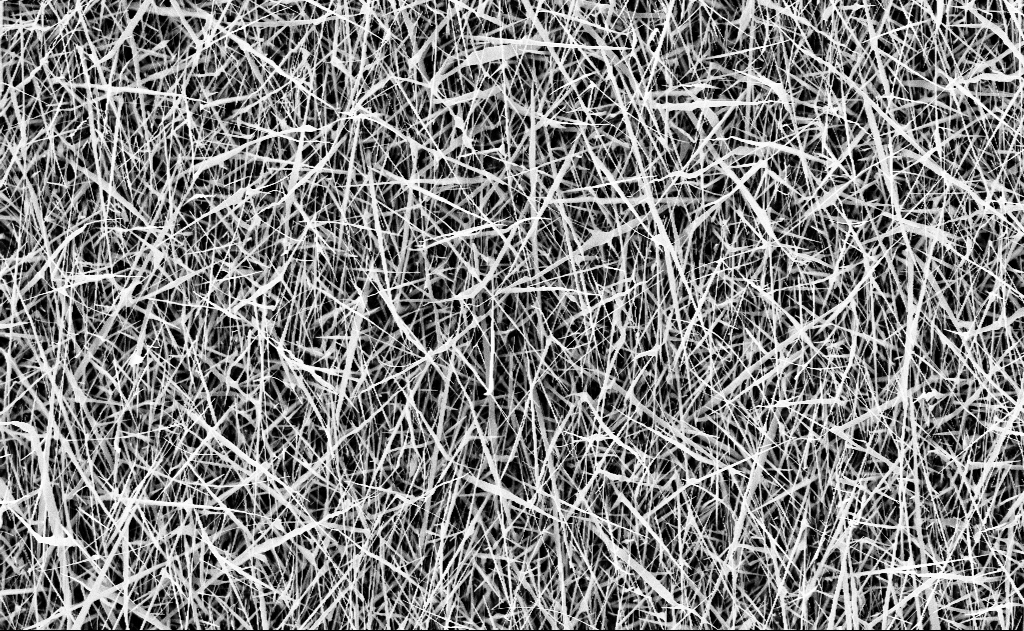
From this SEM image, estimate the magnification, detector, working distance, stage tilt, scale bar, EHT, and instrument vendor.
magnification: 10 K X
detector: InLens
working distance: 17 mm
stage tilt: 0°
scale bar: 2000 nm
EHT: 10 kV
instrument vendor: Zeiss